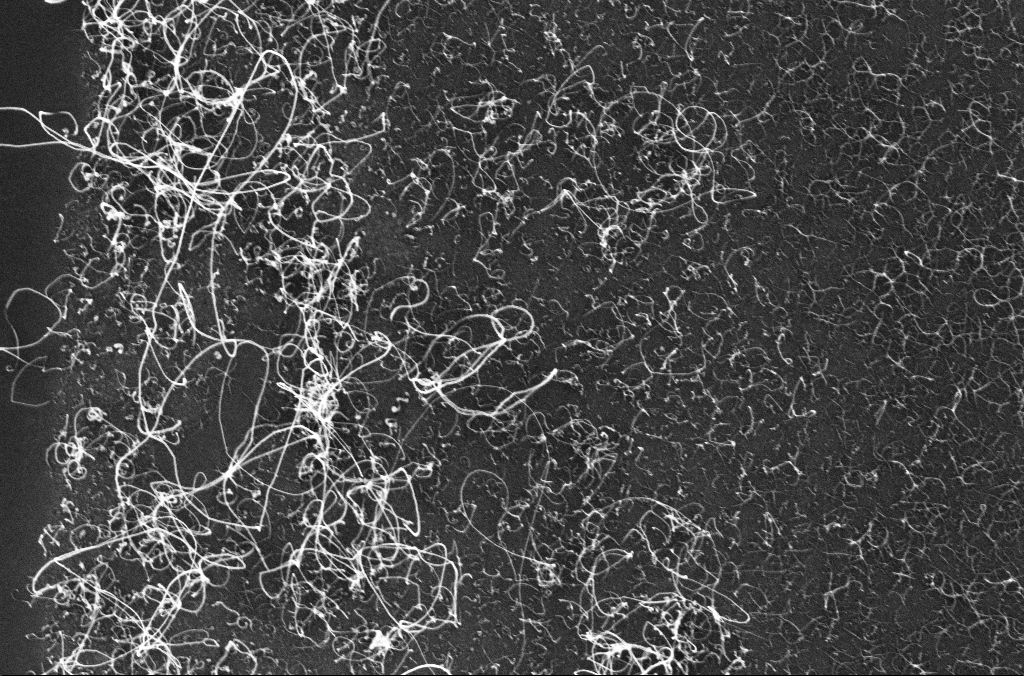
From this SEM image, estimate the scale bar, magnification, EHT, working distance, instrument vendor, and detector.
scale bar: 1000 nm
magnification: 45 K X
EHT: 10 kV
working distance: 3.3 mm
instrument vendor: Zeiss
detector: InLens